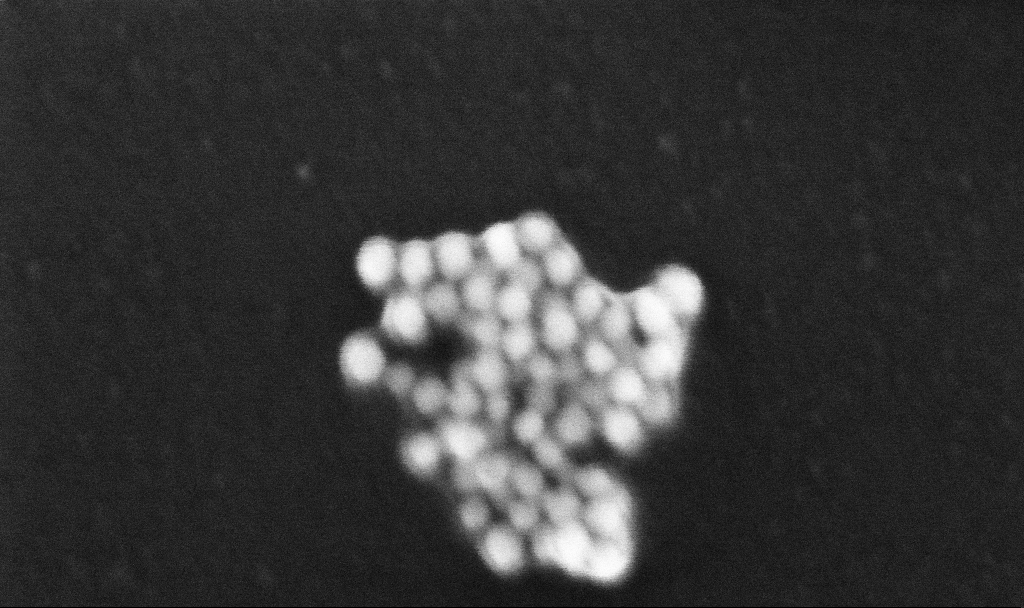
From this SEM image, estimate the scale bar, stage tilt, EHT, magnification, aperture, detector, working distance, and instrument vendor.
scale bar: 100 nm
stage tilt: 0°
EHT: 10 kV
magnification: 623.33 K X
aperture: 30 µm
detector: InLens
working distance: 3.2 mm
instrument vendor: Zeiss